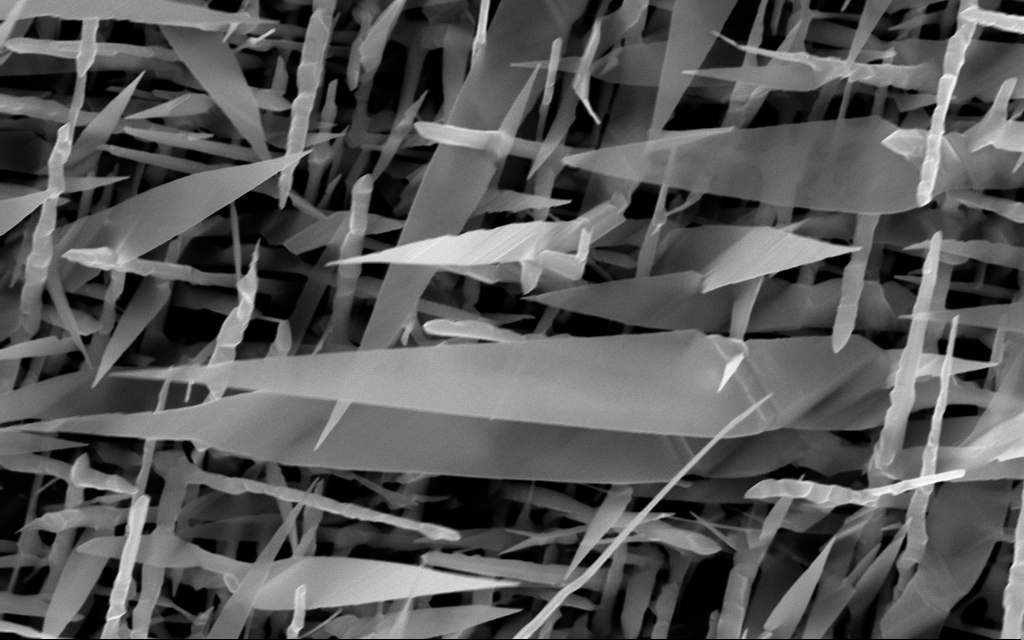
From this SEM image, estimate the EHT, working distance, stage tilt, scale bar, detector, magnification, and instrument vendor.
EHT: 10 kV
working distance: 7 mm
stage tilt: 0°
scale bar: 1000 nm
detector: InLens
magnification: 40 K X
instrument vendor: Zeiss